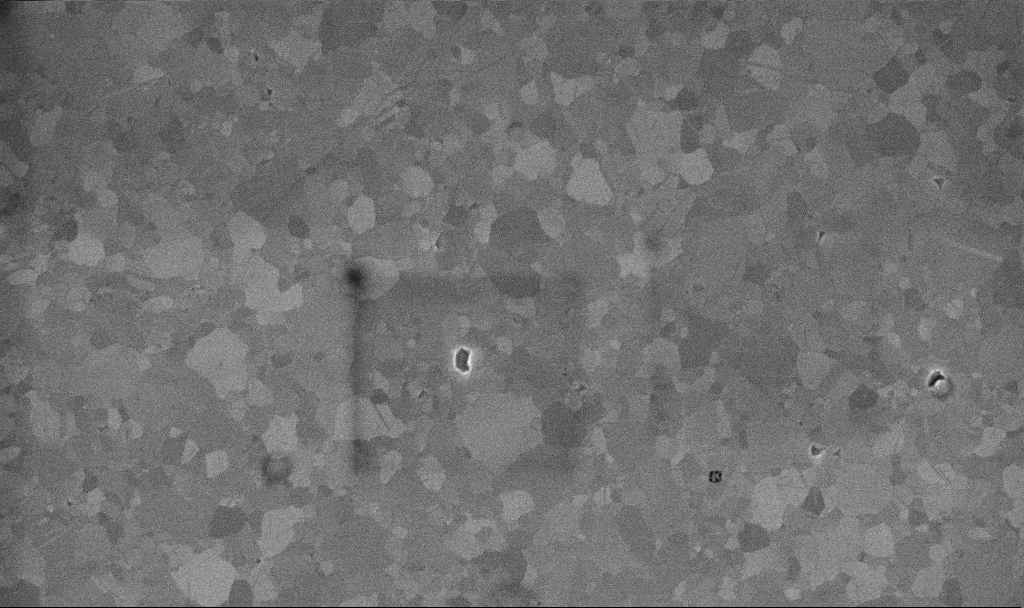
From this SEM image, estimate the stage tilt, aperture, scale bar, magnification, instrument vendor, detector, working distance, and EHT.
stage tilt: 0°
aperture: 30 µm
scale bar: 1000 nm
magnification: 50.87 K X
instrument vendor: Zeiss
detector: InLens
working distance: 3.3 mm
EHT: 10 kV